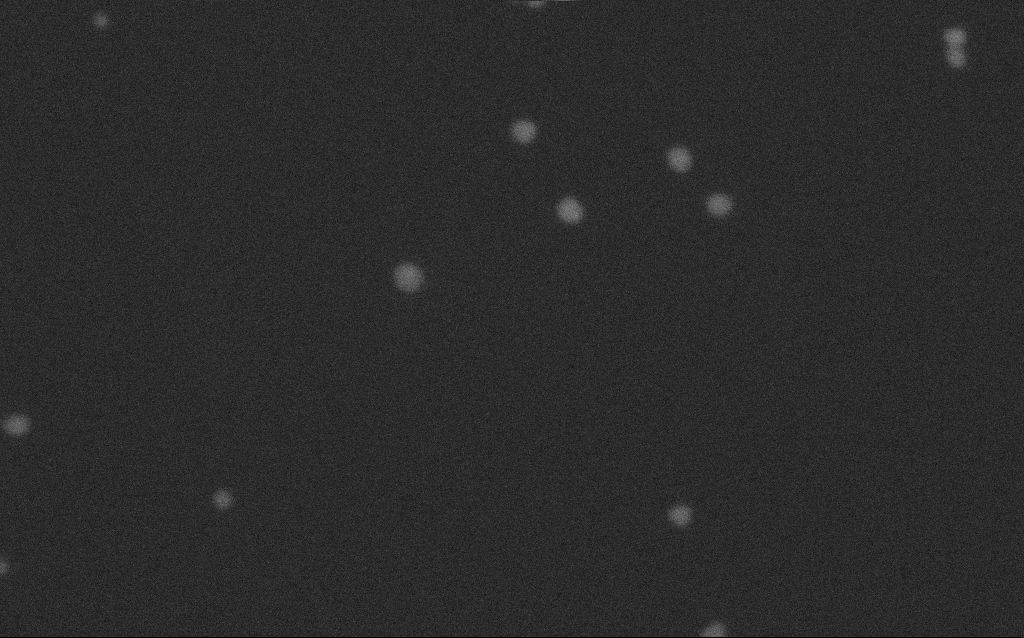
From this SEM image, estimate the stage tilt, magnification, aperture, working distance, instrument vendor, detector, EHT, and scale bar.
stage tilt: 0°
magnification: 673.76 K X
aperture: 30 µm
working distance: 2.7 mm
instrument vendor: Zeiss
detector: SE2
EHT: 8 kV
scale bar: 100 nm